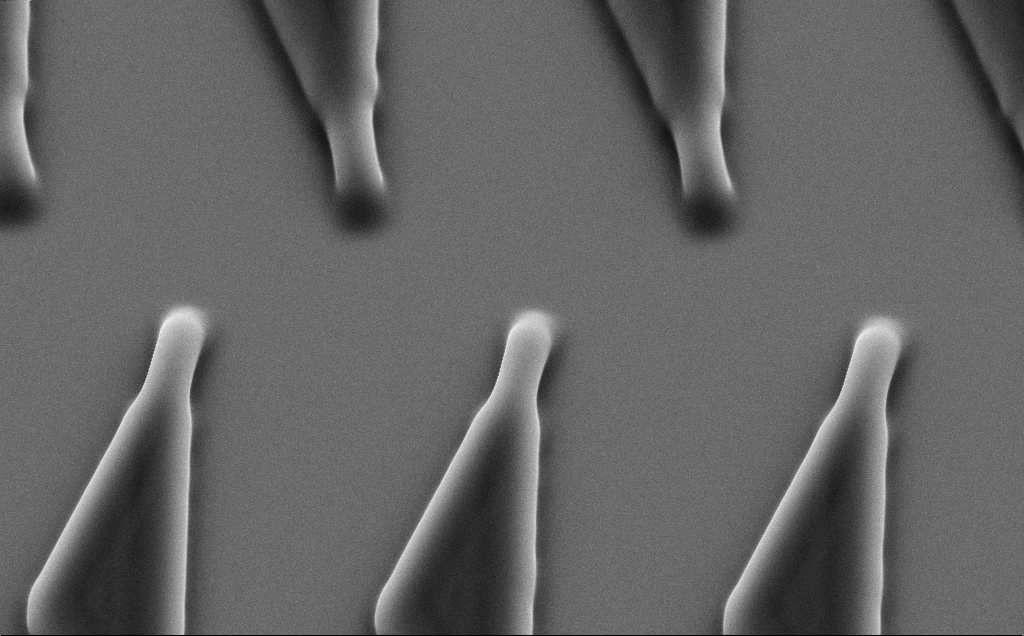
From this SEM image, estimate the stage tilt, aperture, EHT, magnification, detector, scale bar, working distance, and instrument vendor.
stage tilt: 35°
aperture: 30 µm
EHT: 10 kV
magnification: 7.53 K X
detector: SE2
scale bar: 2000 nm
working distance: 8 mm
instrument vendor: Zeiss